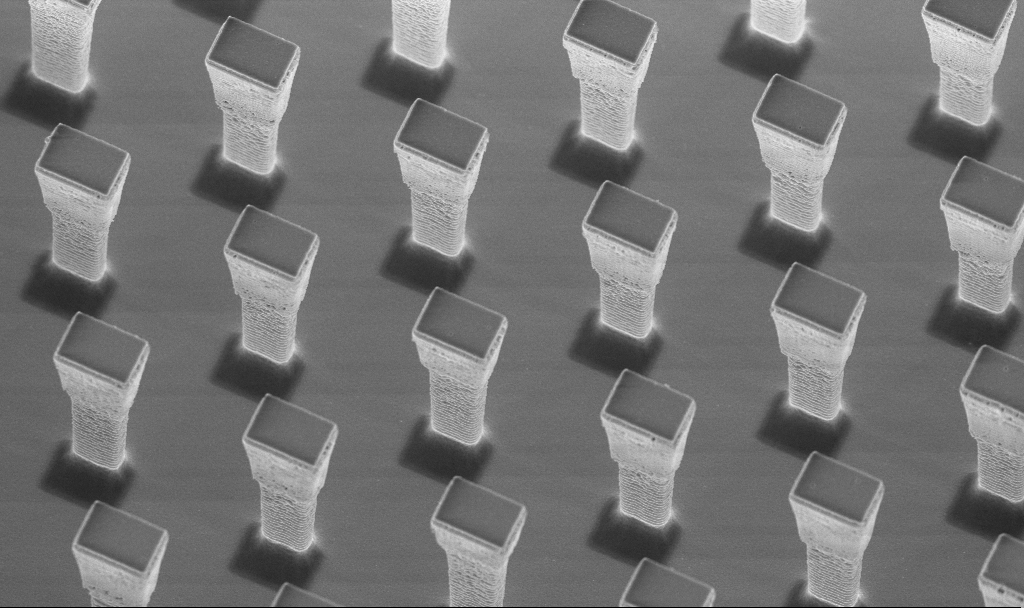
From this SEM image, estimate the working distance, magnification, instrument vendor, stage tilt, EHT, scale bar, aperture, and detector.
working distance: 4.3 mm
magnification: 6.38 K X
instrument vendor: Zeiss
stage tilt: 20°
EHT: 5 kV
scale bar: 10000 nm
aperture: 30 µm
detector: InLens